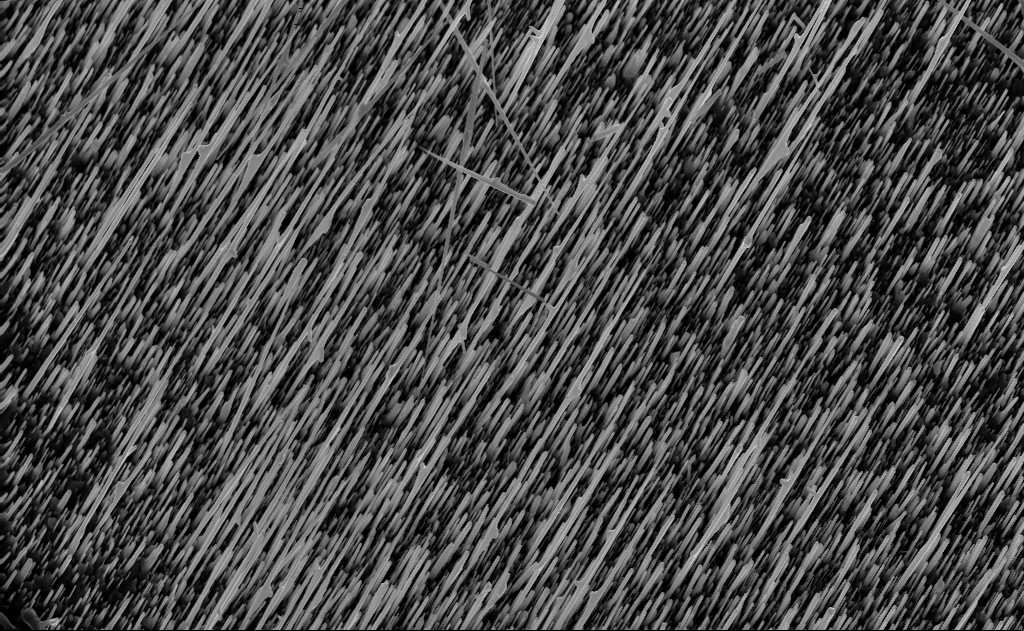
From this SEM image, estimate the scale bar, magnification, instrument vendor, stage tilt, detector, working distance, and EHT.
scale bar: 2000 nm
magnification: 10 K X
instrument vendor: Zeiss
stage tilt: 0°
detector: InLens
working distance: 5 mm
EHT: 10 kV